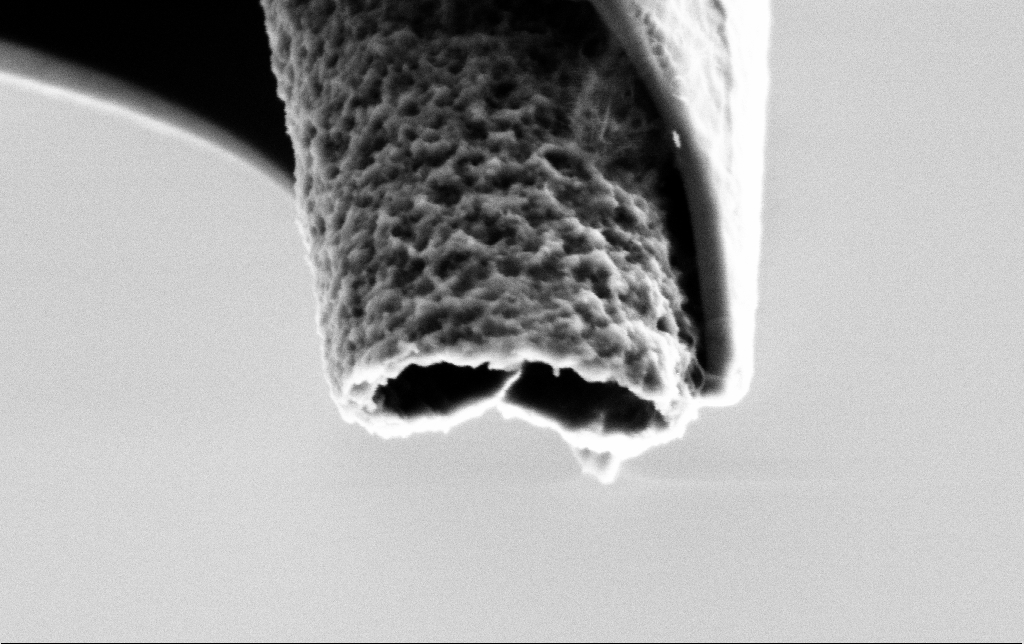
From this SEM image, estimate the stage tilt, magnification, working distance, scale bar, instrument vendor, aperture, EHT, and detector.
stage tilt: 45°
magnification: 100 K X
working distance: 7.4 mm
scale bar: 200 nm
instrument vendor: Zeiss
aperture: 30 µm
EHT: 2 kV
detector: SE2